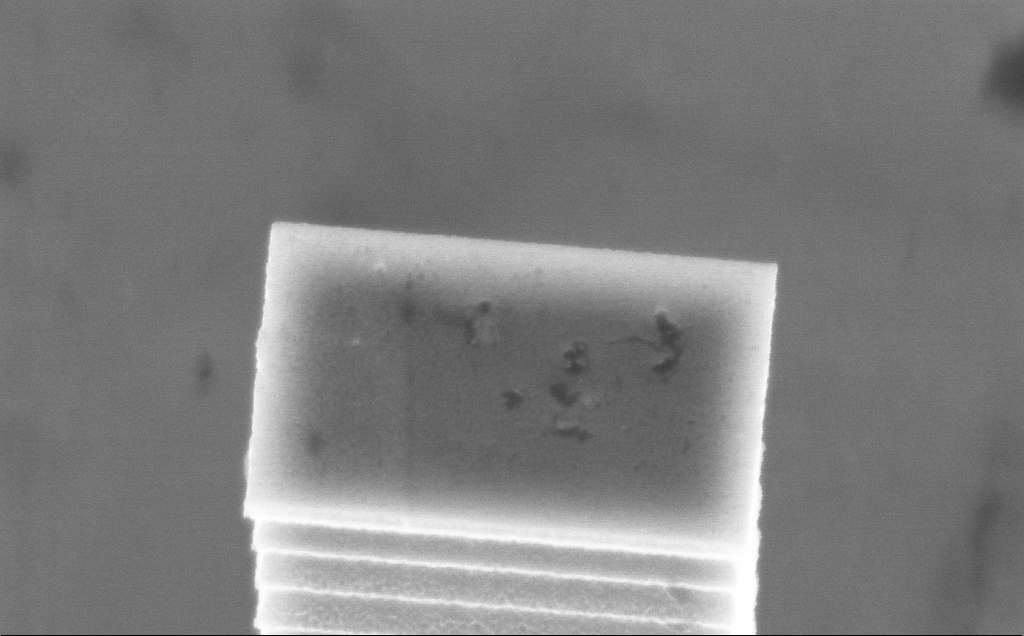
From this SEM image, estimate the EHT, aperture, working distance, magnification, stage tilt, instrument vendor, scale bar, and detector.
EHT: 7.5 kV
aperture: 30 µm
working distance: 5 mm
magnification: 38.76 K X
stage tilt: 45°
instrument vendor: Zeiss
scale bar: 1000 nm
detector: InLens